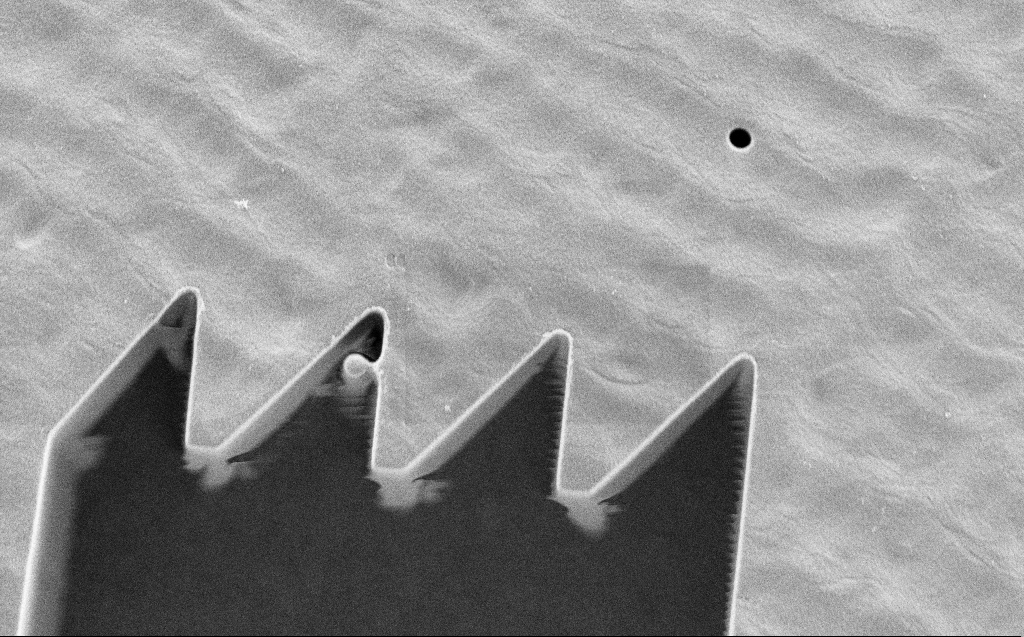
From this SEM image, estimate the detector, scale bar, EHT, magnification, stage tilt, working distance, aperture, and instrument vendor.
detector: SE2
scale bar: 10000 nm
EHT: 1 kV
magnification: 6.01 K X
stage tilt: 0°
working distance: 5 mm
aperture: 30 µm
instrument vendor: Zeiss